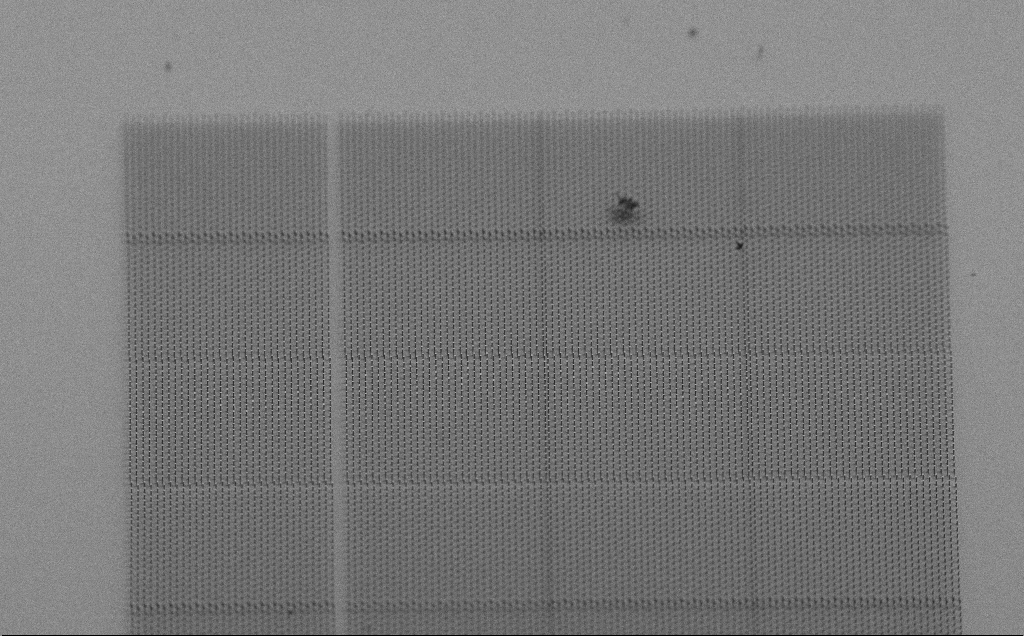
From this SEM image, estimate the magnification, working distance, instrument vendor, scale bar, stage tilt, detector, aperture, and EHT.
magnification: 0.26 K X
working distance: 7 mm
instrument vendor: Zeiss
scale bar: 100000 nm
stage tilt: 60°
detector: SE2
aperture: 30 µm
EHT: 3 kV